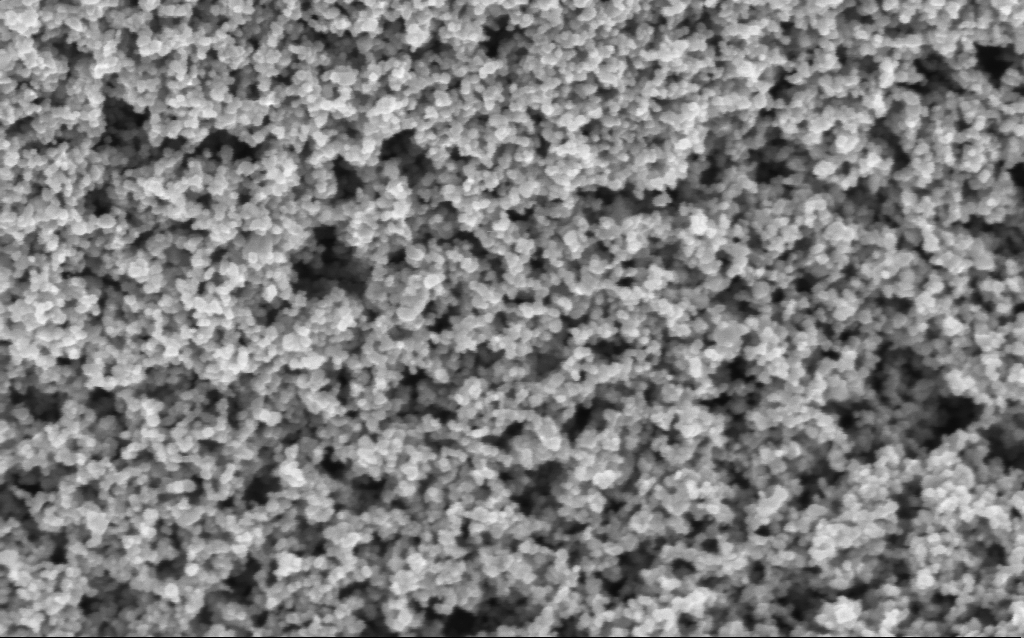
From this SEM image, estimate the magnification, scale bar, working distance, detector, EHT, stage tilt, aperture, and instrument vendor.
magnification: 130 K X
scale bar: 100 nm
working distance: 7.6 mm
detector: InLens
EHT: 3 kV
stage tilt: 0°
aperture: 30 µm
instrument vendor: Zeiss